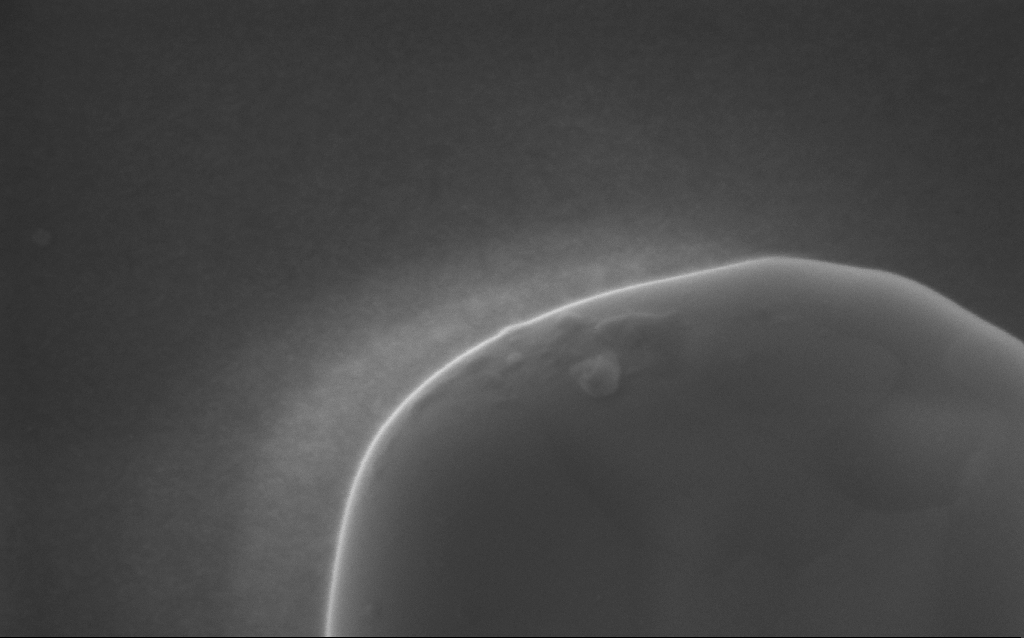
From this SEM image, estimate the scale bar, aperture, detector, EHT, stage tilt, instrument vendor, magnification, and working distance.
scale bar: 100 nm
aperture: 30 µm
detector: InLens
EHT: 5 kV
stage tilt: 0°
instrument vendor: Zeiss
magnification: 350.53 K X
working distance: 3 mm